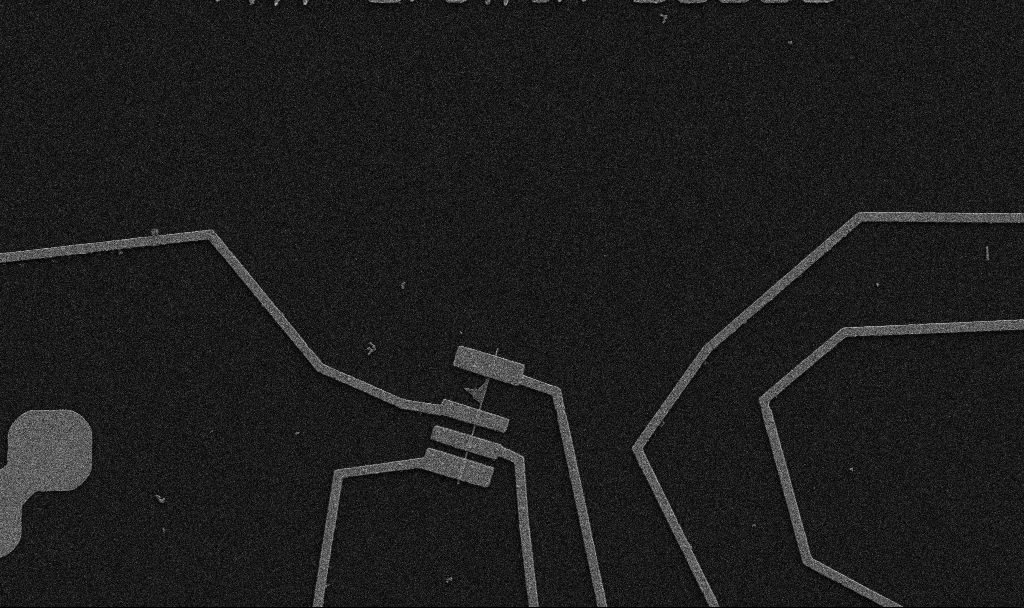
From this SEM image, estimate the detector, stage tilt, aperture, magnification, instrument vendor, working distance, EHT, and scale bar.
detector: SE2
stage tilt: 0°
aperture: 30 µm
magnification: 5 K X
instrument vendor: Zeiss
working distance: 10.7 mm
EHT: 5 kV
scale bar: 10000 nm